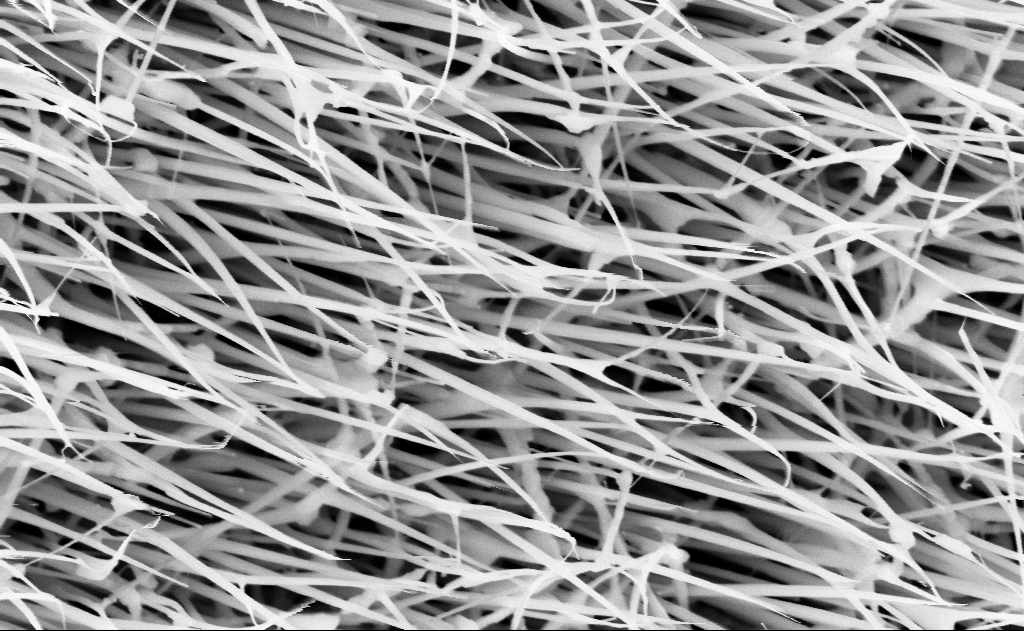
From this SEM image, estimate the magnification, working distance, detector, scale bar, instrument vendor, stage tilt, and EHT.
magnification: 60 K X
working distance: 13 mm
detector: InLens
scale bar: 1000 nm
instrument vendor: Zeiss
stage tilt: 0°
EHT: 10 kV